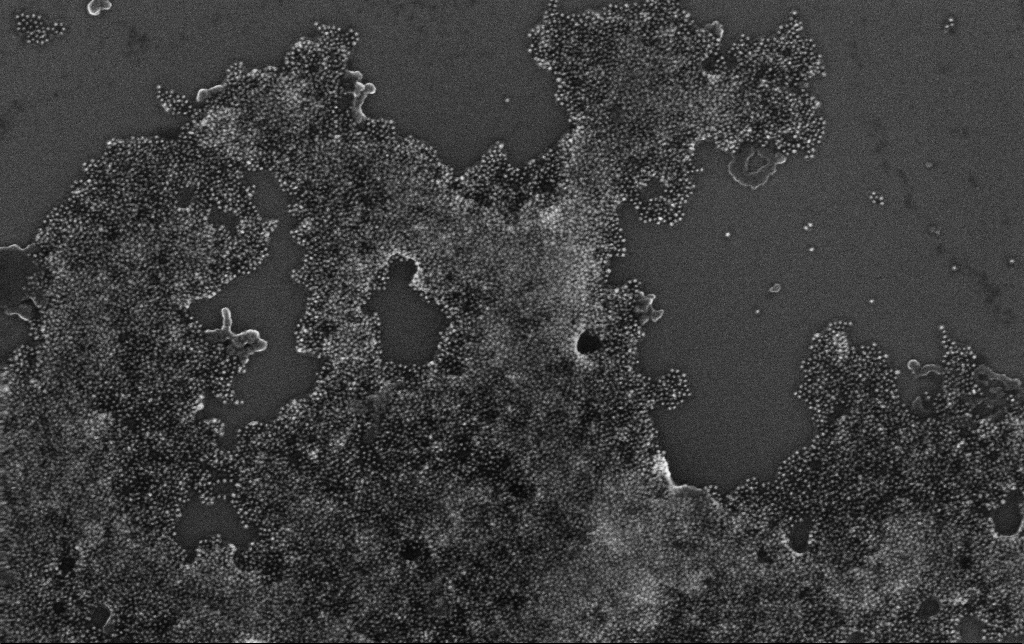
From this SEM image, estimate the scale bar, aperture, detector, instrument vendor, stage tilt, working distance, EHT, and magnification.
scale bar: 200 nm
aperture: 30 µm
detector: InLens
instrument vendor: Zeiss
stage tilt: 0°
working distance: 3.4 mm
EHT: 10 kV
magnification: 100 K X